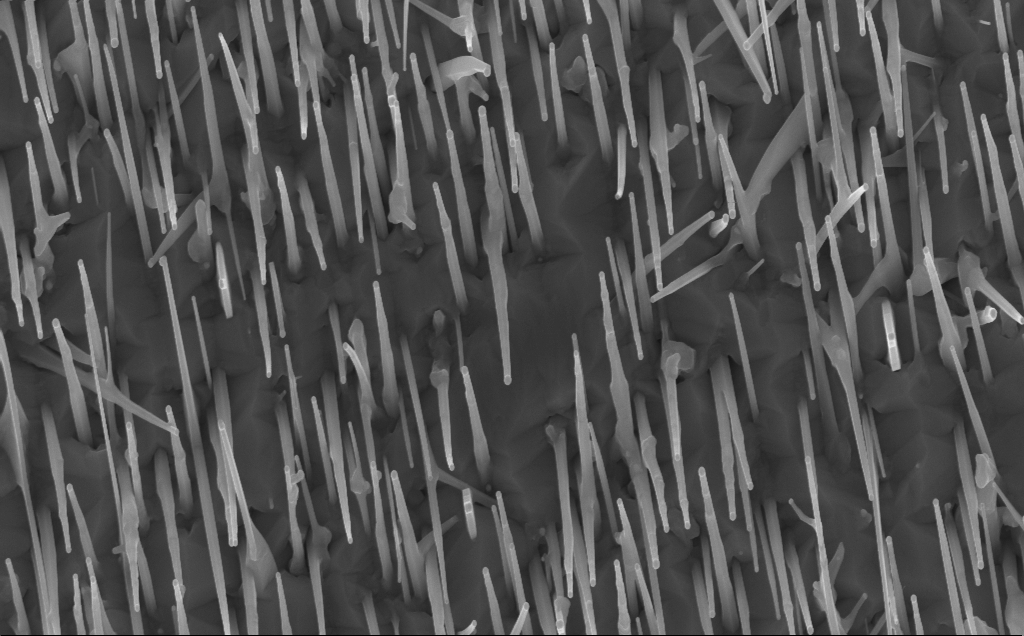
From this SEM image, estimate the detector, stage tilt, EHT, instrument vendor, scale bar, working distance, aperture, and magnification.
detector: InLens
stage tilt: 0°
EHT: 10 kV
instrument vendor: Zeiss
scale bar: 1000 nm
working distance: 7 mm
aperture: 30 µm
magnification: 40 K X